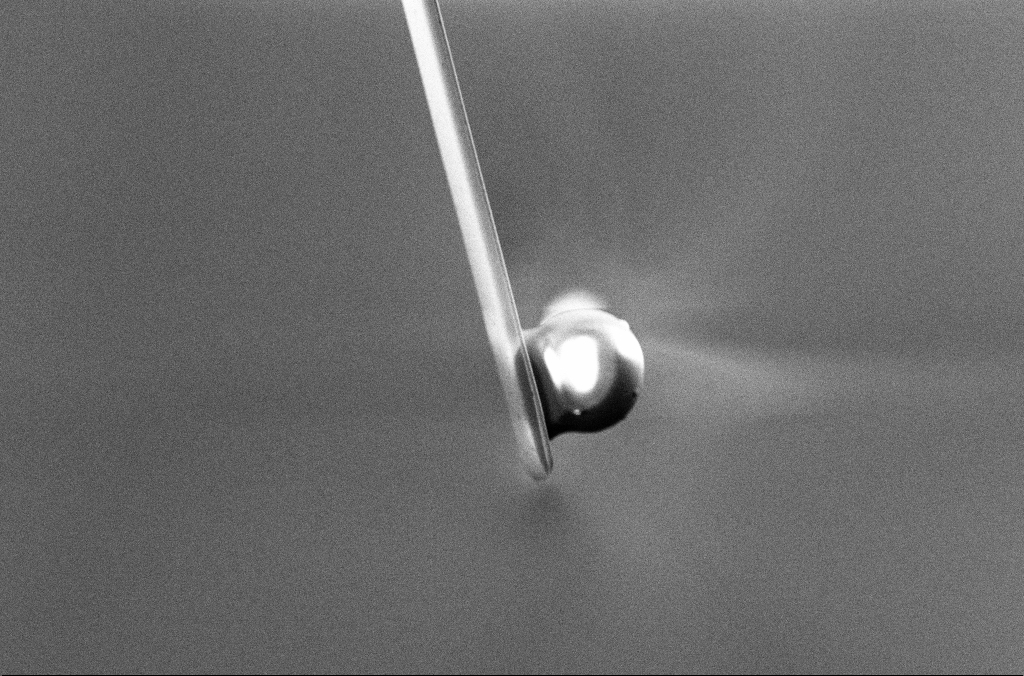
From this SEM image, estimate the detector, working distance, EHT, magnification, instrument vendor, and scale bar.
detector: InLens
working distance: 5.3 mm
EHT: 5 kV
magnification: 2.5 K X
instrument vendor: Zeiss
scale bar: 10000 nm